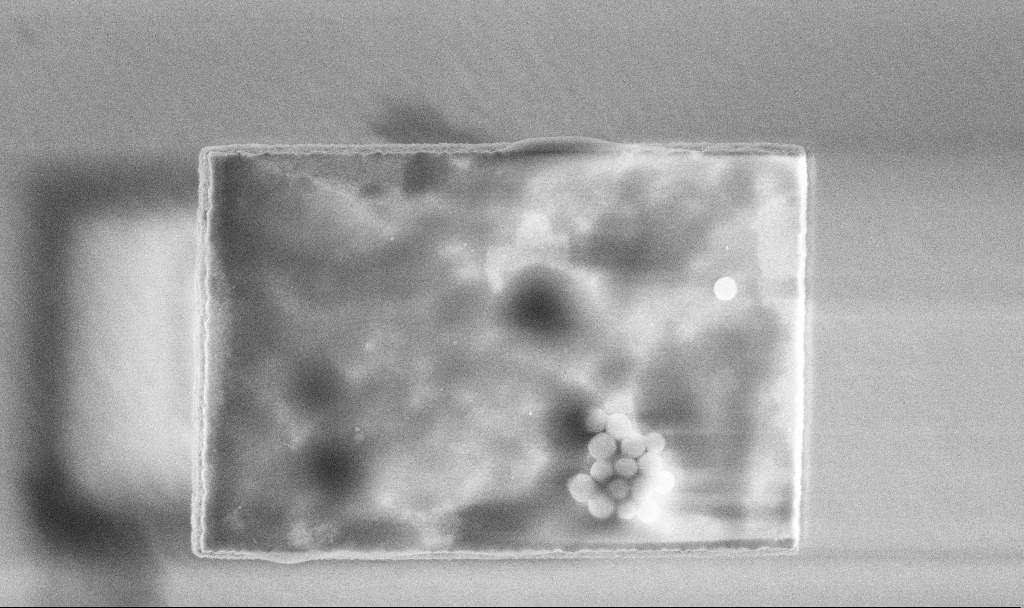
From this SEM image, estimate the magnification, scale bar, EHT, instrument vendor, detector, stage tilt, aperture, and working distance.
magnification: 50 K X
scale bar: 1000 nm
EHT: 3 kV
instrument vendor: Zeiss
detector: InLens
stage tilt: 0°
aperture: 30 µm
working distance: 3.3 mm